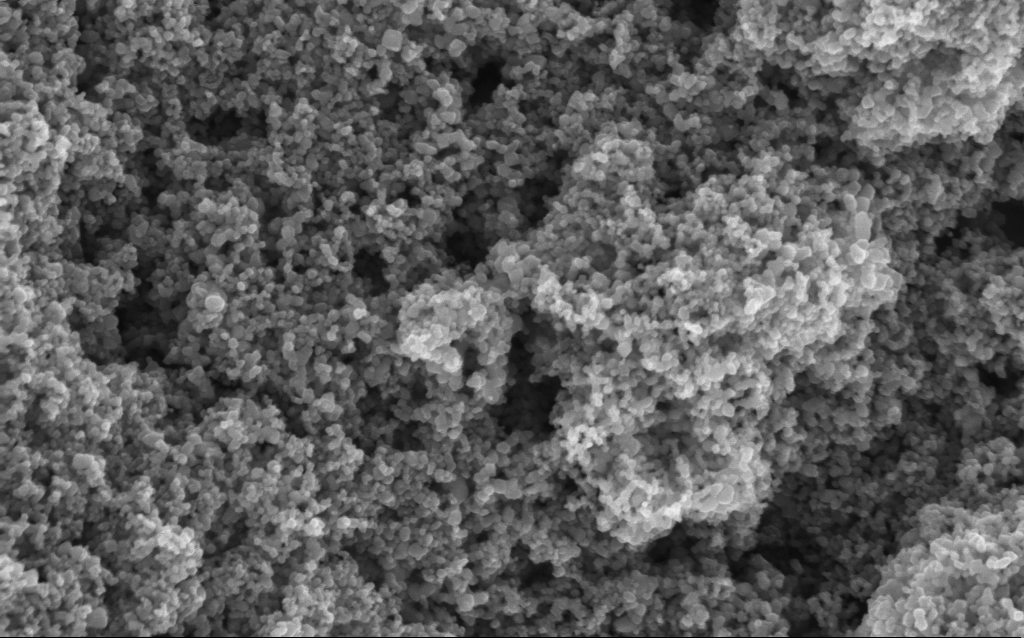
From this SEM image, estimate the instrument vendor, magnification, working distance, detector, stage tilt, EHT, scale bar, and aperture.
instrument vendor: Zeiss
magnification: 114.56 K X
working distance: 4.2 mm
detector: InLens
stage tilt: -0°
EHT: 5 kV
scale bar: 200 nm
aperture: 30 µm